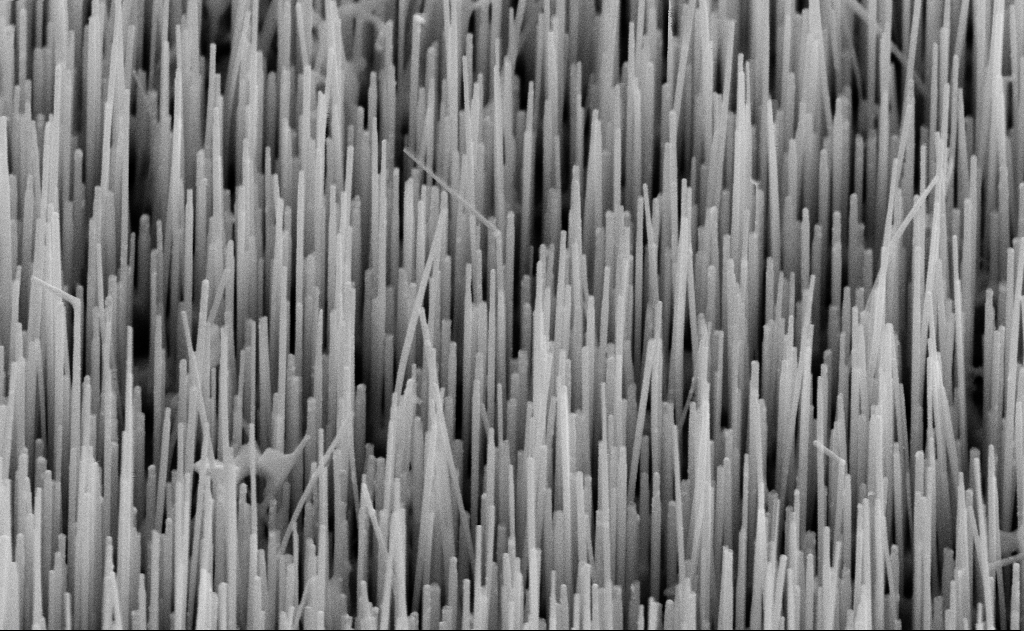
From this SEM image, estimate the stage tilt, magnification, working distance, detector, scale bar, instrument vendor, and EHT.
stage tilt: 45°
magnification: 60 K X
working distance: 11 mm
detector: SE2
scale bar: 1000 nm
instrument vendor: Zeiss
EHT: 10 kV